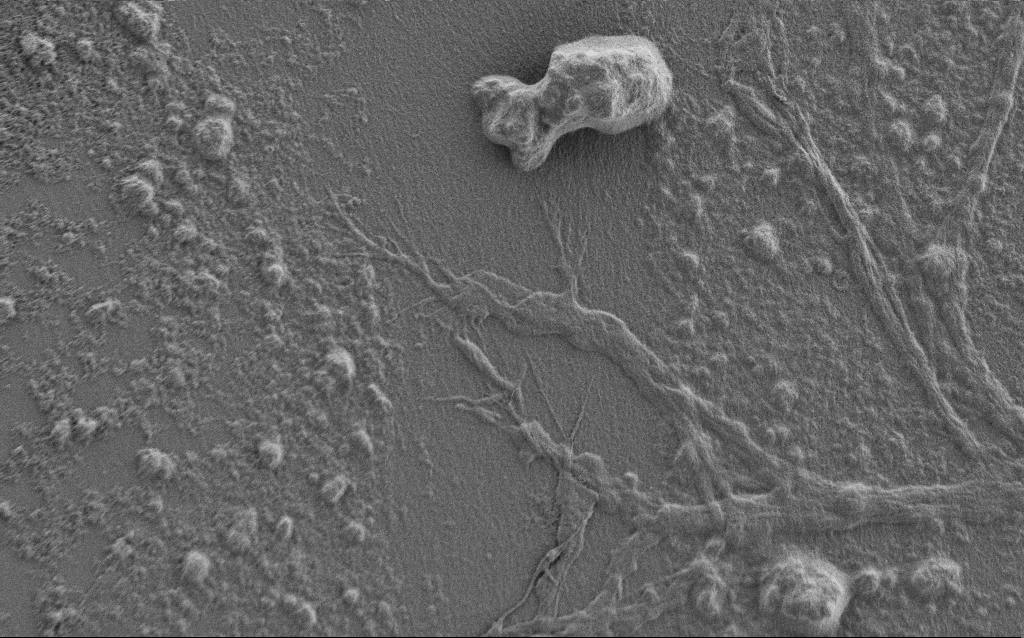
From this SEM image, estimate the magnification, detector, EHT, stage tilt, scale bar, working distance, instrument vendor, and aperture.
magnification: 4 K X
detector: SE2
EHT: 0.9 kV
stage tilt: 0°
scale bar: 10000 nm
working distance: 7 mm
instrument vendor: Zeiss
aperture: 30 µm